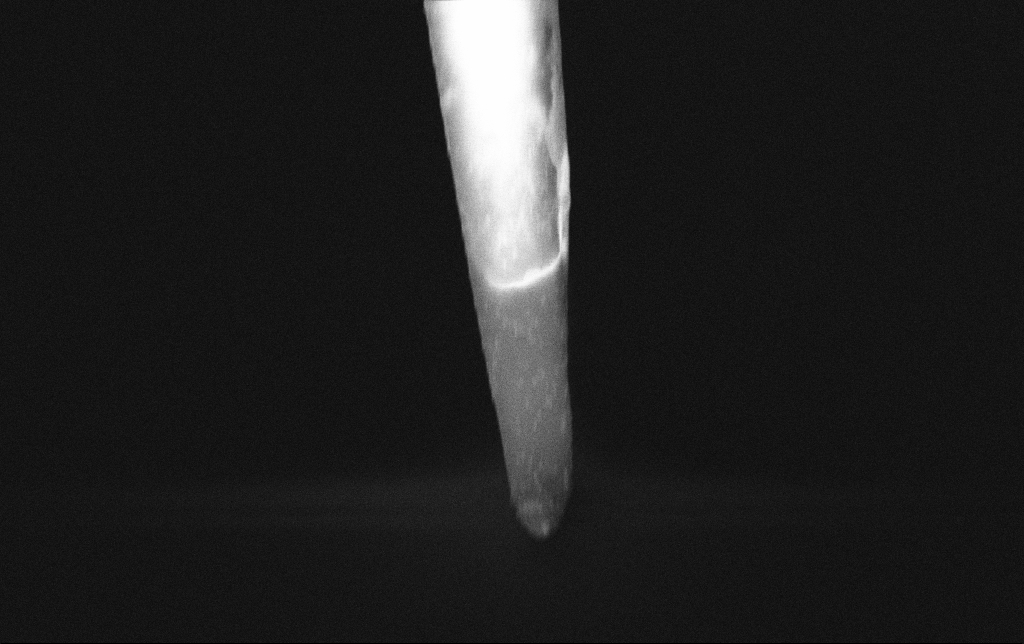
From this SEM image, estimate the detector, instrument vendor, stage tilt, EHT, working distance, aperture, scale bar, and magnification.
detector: InLens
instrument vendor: Zeiss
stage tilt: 0°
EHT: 3 kV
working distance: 6.5 mm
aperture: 30 µm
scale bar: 2000 nm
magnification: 20 K X